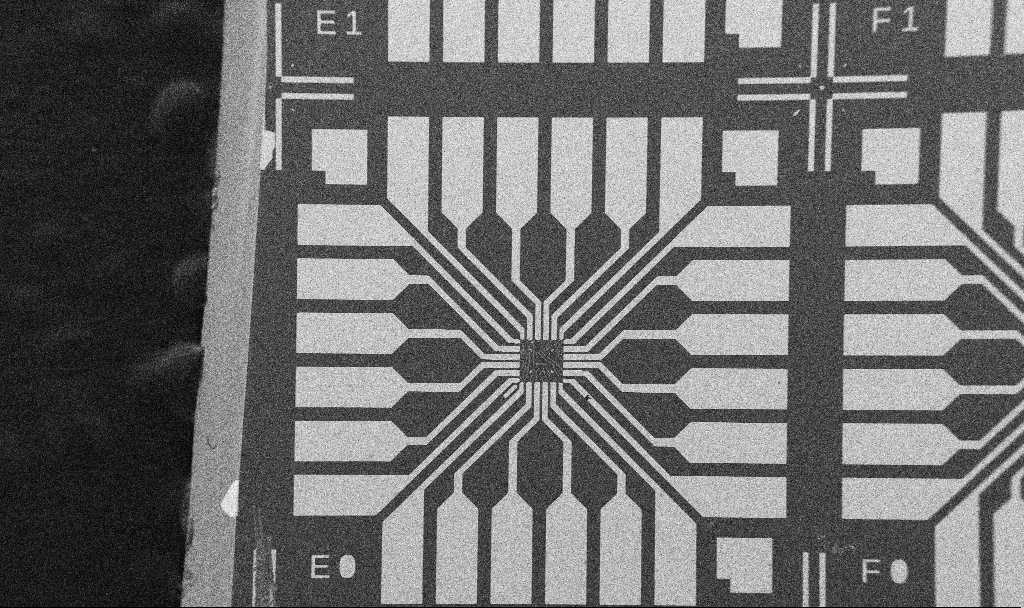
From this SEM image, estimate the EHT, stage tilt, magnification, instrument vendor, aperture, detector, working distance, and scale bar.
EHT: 5 kV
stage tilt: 0°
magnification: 0.1 K X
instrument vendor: Zeiss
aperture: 30 µm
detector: SE2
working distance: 10.7 mm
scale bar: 200000 nm